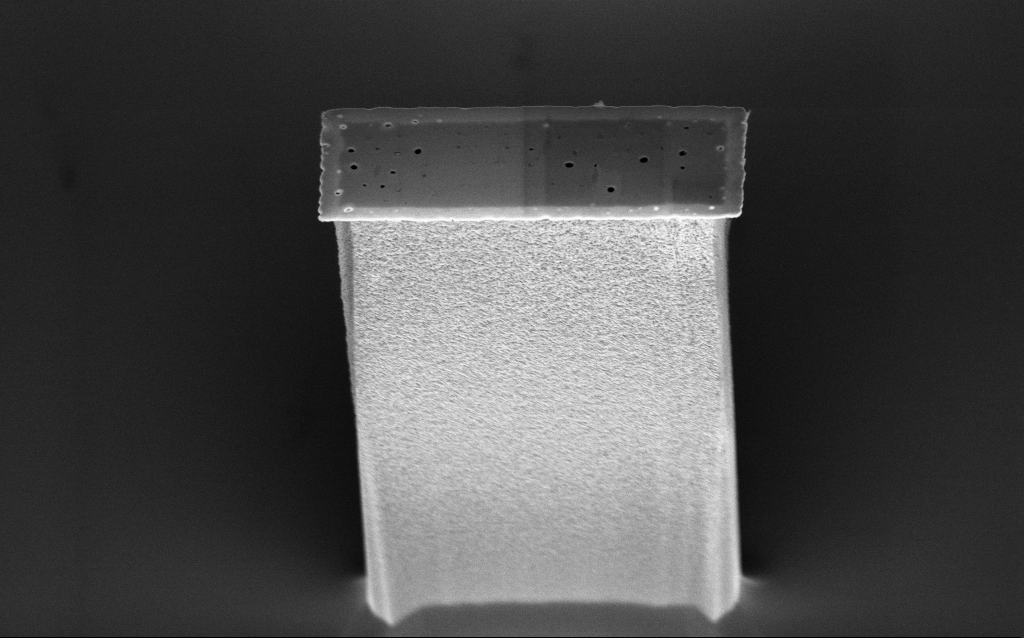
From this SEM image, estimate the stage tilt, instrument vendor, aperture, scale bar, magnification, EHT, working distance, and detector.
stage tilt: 45°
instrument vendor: Zeiss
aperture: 30 µm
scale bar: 1000 nm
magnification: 19.52 K X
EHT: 3 kV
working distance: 6.2 mm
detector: InLens